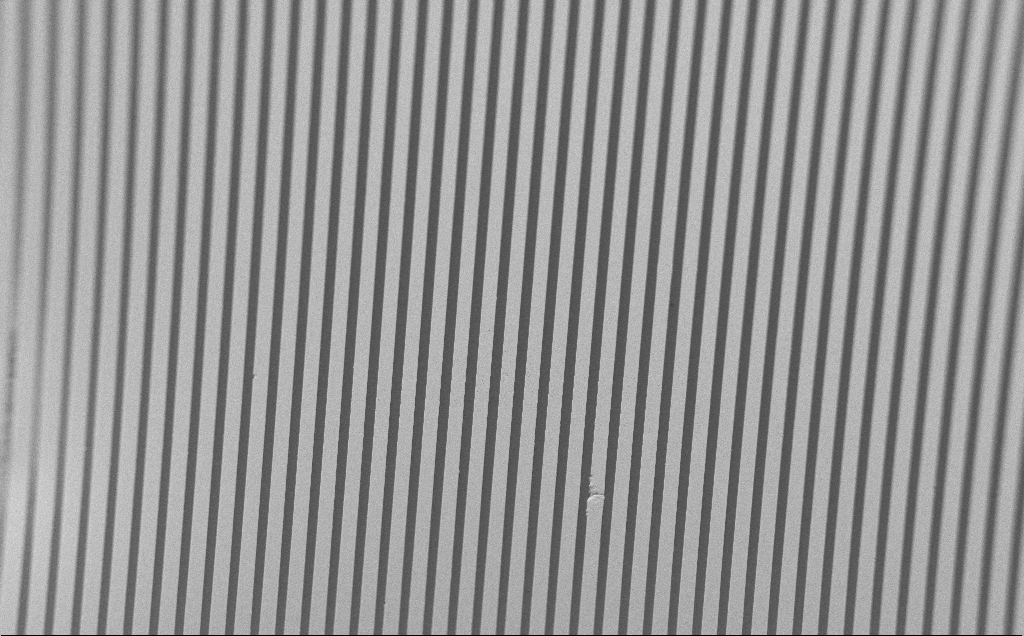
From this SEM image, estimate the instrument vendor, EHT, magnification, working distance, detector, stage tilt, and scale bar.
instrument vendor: Zeiss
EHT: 1.2 kV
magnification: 0.28 K X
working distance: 6 mm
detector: SE2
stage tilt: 45°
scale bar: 100000 nm